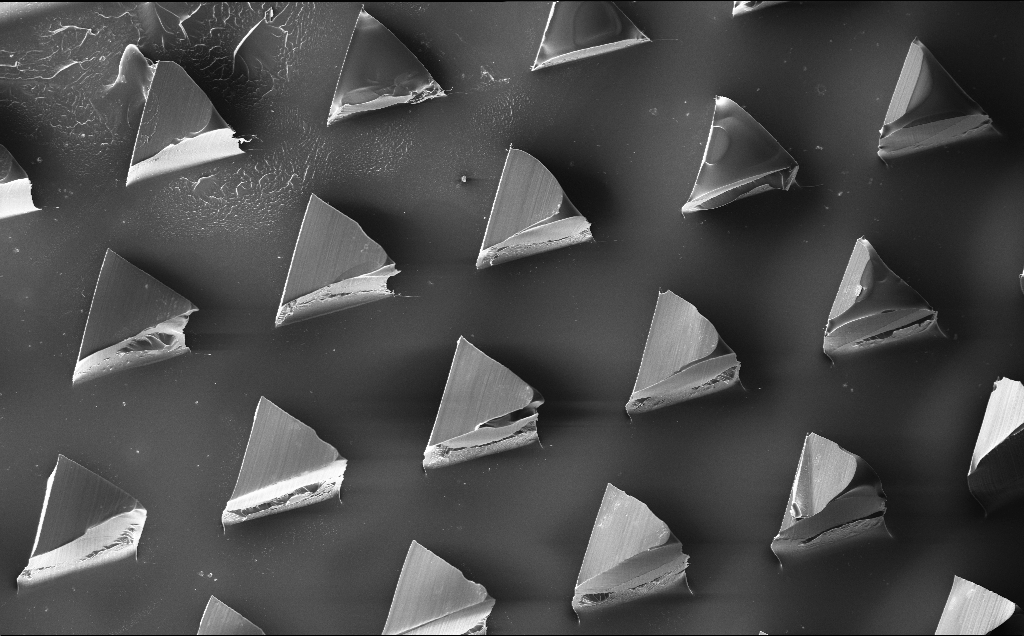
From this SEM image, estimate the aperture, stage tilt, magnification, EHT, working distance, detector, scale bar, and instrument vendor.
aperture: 30 µm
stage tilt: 0°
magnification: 0.167 K X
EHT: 10 kV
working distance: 9 mm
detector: InLens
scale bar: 200000 nm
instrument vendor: Zeiss